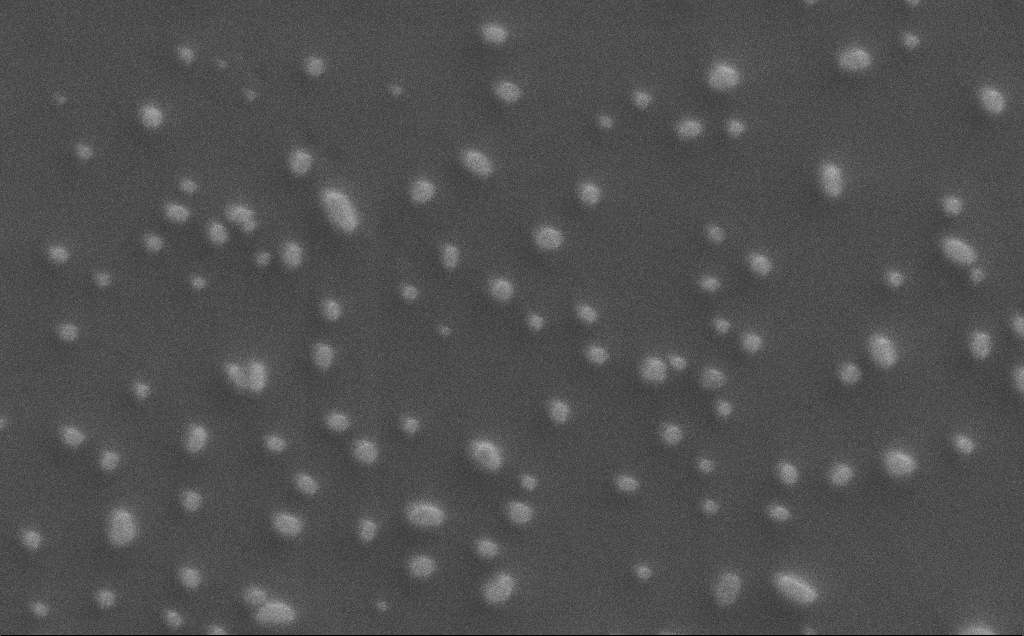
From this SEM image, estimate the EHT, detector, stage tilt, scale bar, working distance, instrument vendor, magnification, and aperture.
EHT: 0.5 kV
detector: SE2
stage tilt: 0°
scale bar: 2000 nm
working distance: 3 mm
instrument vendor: Zeiss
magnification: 20 K X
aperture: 30 µm